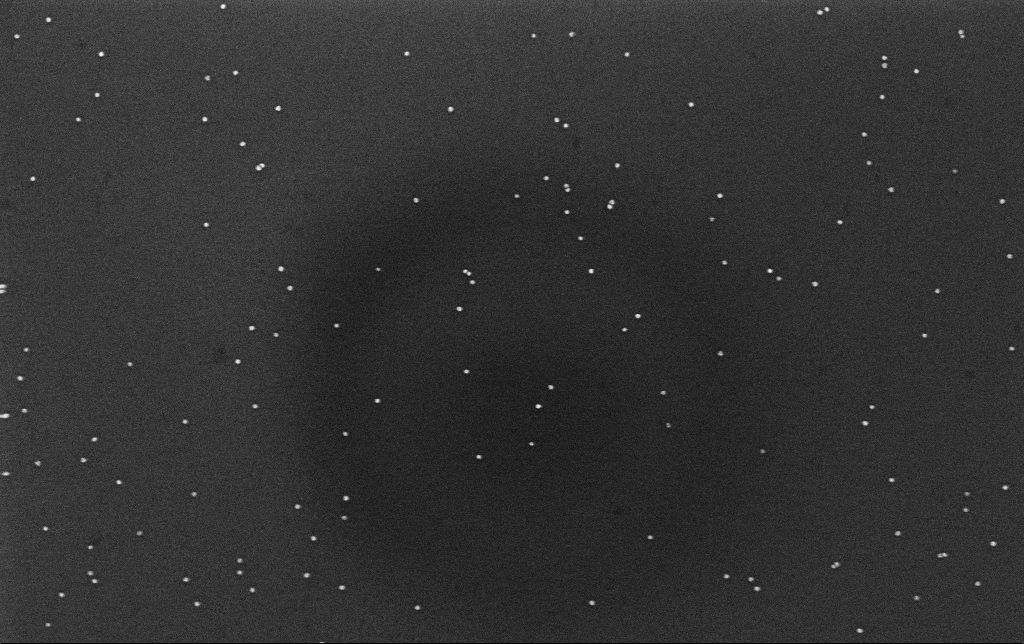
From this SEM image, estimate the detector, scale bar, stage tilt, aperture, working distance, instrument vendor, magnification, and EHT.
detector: InLens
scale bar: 200 nm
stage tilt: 0°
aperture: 30 µm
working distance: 3.1 mm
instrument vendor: Zeiss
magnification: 100 K X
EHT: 10 kV